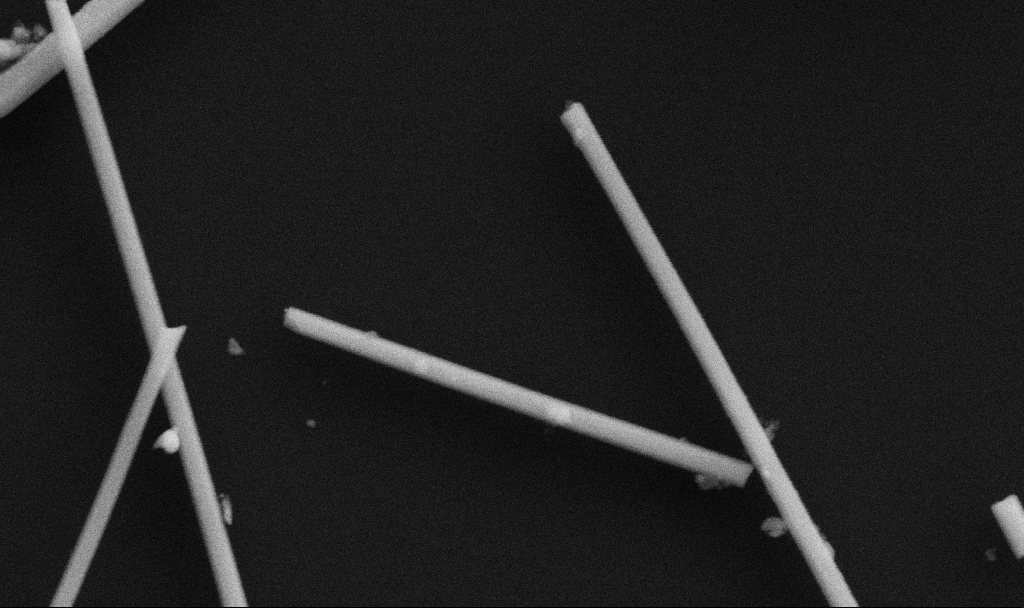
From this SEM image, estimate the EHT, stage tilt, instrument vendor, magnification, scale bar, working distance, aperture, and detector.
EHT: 5 kV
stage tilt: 0°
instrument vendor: Zeiss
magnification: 108.15 K X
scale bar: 200 nm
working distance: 6.7 mm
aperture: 30 µm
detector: SE2